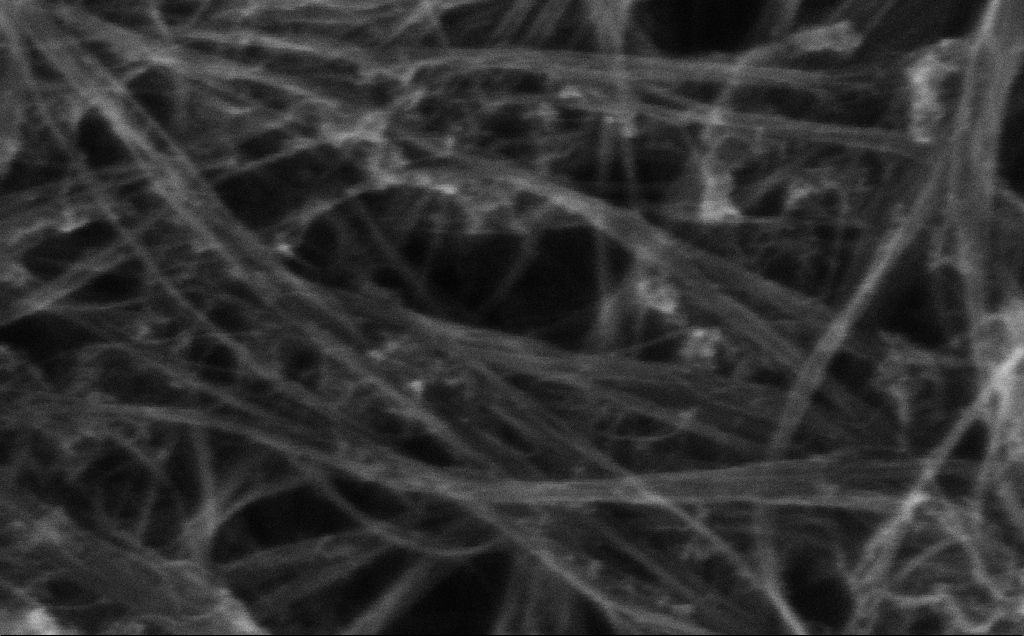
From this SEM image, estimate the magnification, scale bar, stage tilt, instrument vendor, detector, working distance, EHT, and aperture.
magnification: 325.16 K X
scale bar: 200 nm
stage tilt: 0°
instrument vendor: Zeiss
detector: InLens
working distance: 3 mm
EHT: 10 kV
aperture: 30 µm